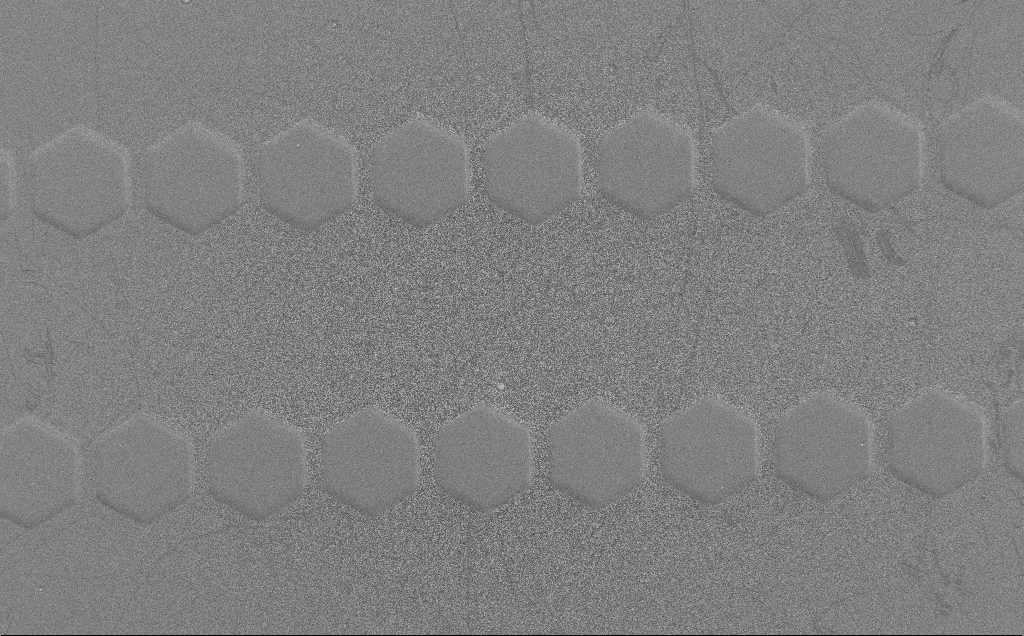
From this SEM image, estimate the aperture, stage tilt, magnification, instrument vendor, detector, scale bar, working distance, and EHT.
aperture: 30 µm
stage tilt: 0°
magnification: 0.698 K X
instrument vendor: Zeiss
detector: SE2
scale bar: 100000 nm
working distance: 4 mm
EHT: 1.5 kV